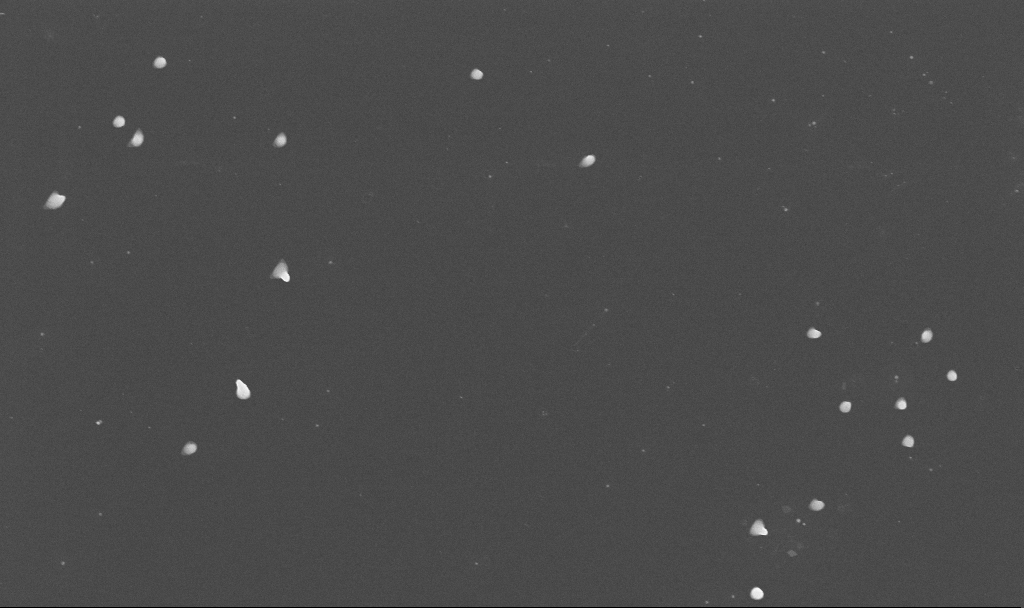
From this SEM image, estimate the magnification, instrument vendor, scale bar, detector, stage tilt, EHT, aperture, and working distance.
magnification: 50 K X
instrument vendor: Zeiss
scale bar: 1000 nm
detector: InLens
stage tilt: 0°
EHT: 10 kV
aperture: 30 µm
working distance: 3.4 mm